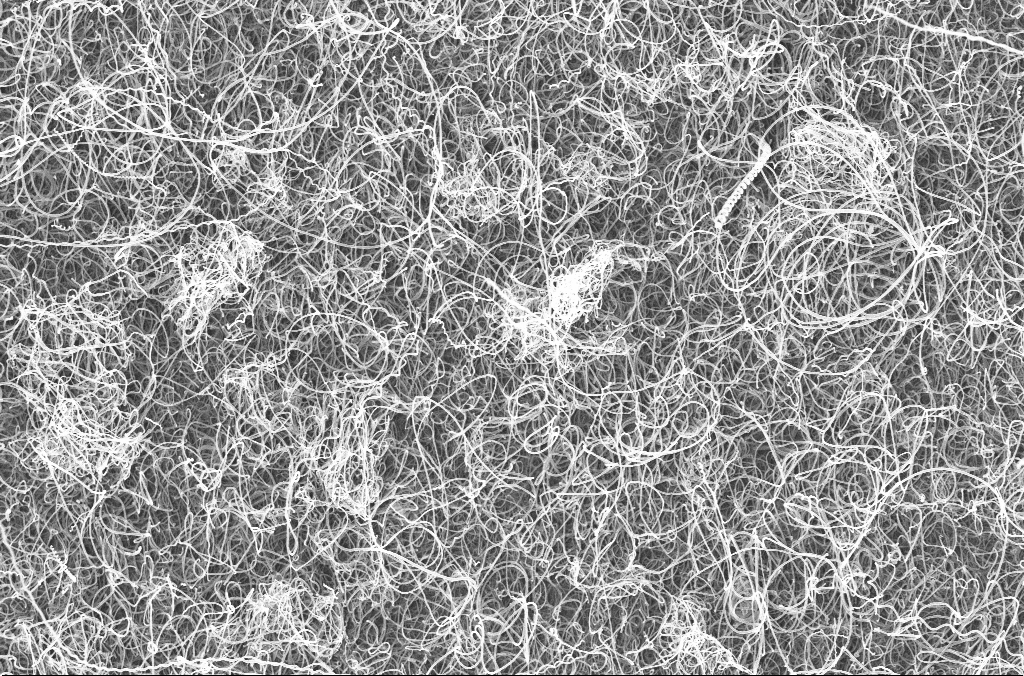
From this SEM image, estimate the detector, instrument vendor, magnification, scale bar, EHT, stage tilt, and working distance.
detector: InLens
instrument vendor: Zeiss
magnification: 15 K X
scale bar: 1000 nm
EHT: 20 kV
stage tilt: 0°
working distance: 4.2 mm